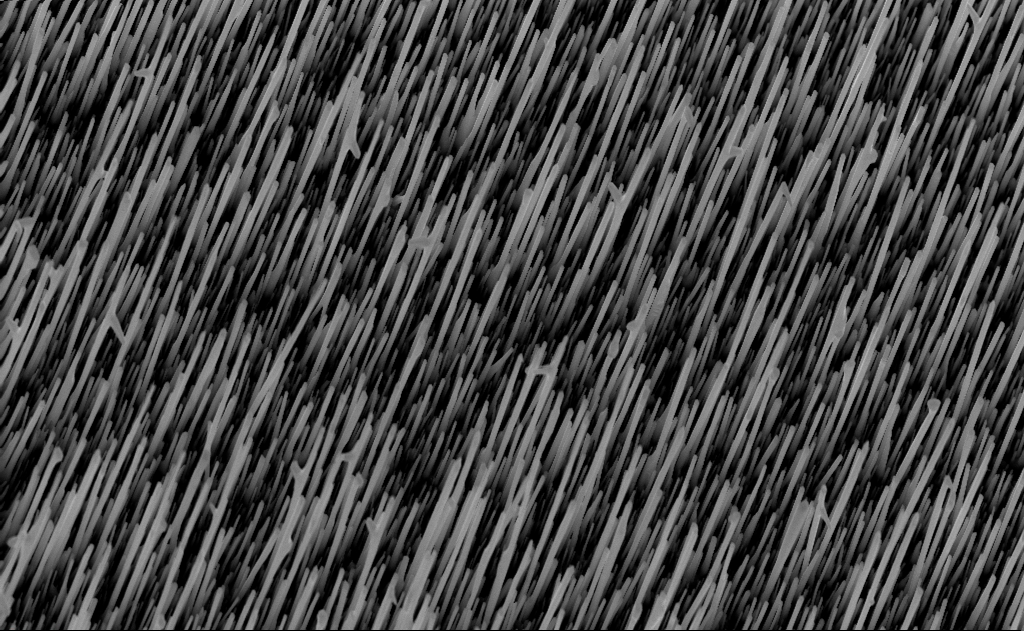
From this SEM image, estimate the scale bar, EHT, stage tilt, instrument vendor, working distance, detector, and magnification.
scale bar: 2000 nm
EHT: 10 kV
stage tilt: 0°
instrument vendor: Zeiss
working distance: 8 mm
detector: InLens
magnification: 20 K X